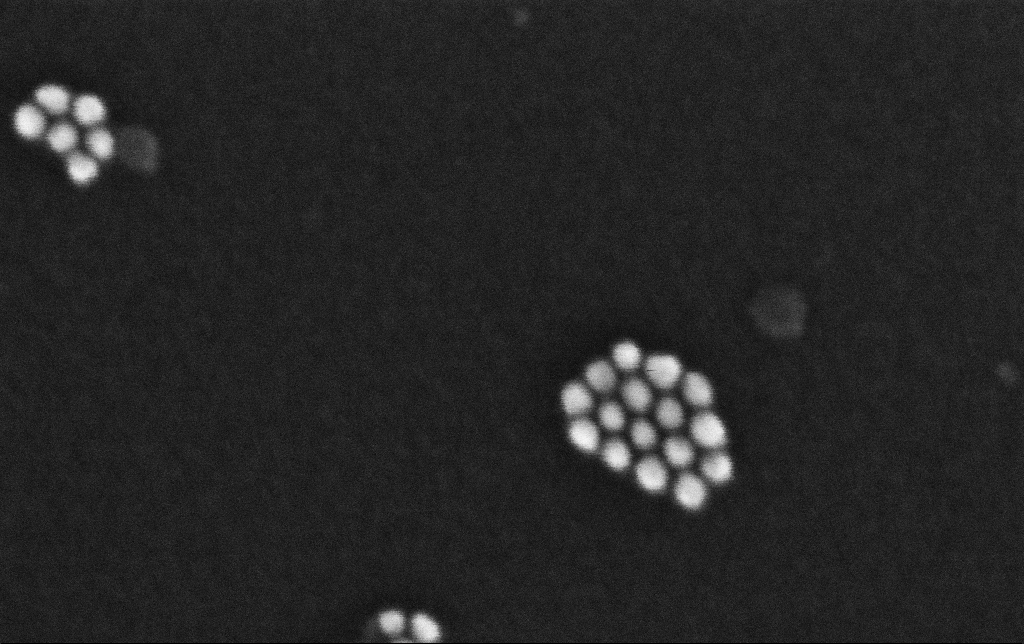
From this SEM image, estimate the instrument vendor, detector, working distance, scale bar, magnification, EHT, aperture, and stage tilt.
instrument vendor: Zeiss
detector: InLens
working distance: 3.4 mm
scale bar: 20 nm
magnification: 881.52 K X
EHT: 10 kV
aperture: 30 µm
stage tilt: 0°